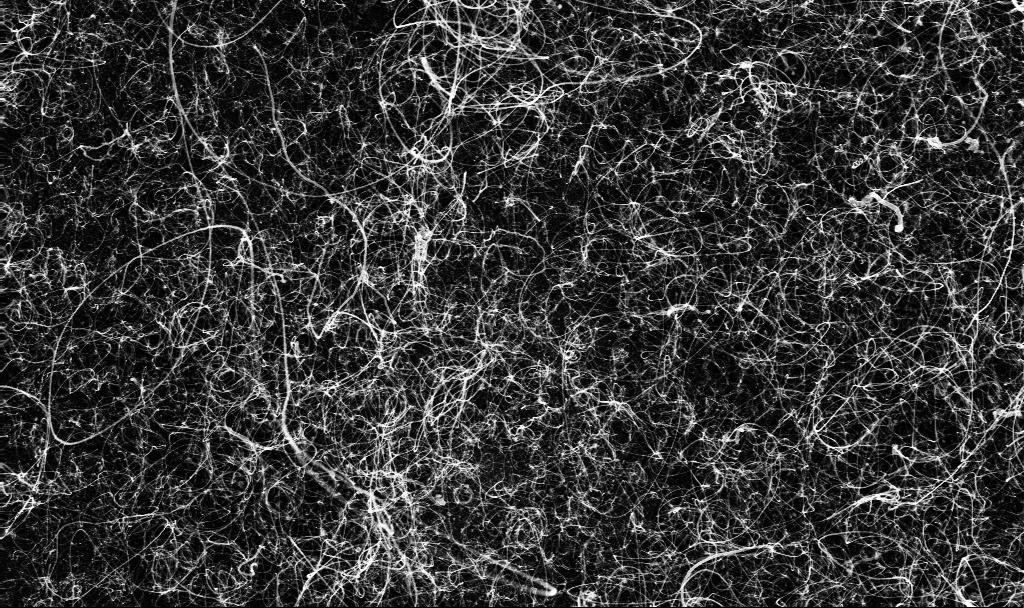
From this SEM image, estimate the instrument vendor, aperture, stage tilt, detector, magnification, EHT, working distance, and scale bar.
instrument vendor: Zeiss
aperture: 30 µm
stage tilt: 0°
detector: InLens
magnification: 15.72 K X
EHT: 10 kV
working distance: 2.7 mm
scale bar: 1000 nm